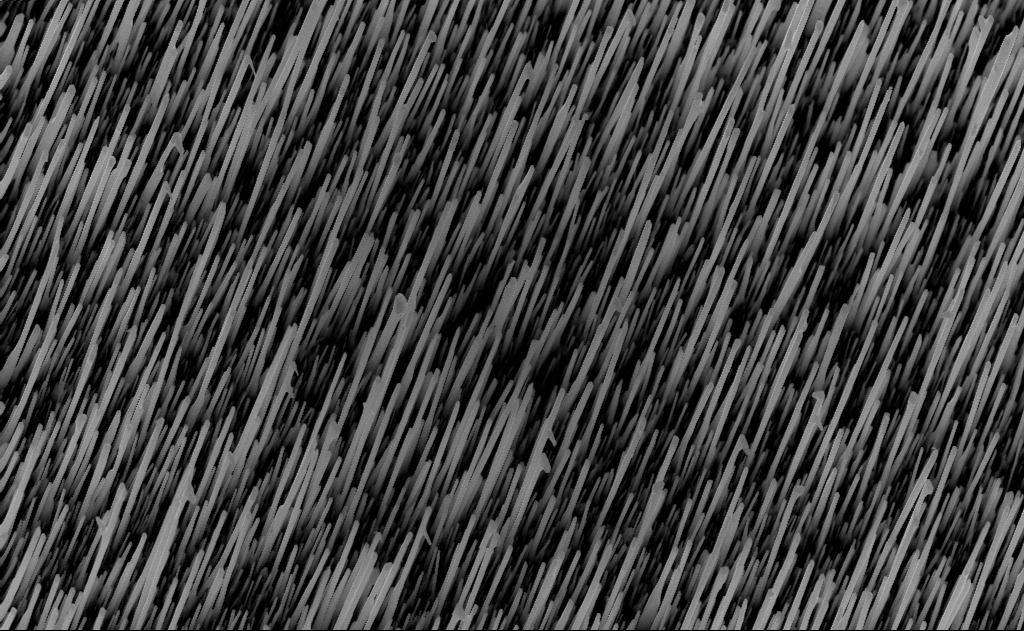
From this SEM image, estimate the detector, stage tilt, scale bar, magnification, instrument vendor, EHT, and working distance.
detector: InLens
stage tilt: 0°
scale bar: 2000 nm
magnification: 20 K X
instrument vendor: Zeiss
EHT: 10 kV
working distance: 7 mm